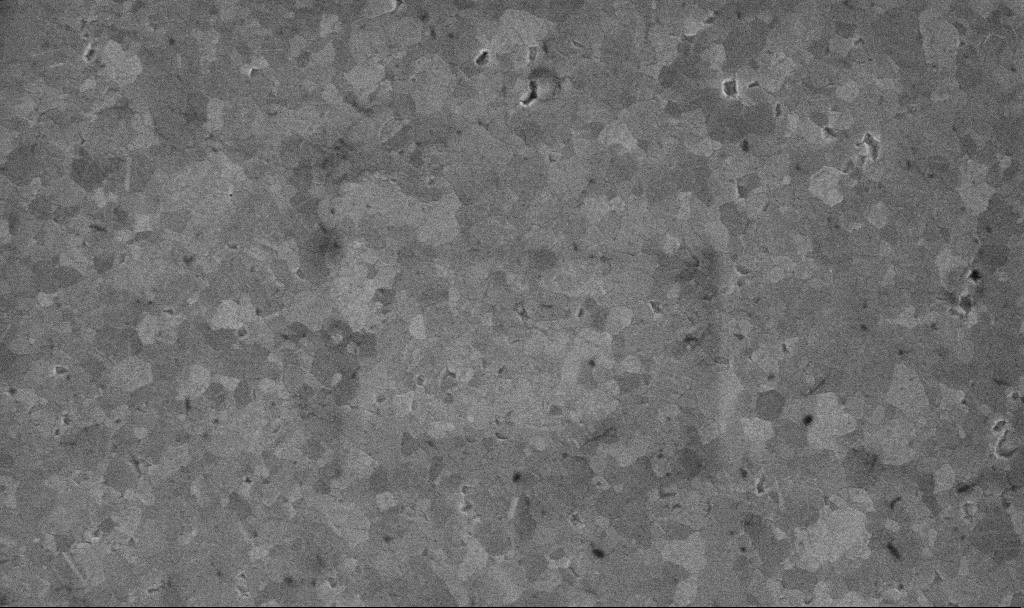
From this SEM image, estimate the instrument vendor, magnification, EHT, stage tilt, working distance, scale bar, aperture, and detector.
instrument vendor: Zeiss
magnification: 46.56 K X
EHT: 10 kV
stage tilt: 0°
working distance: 3.1 mm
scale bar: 1000 nm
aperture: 30 µm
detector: InLens